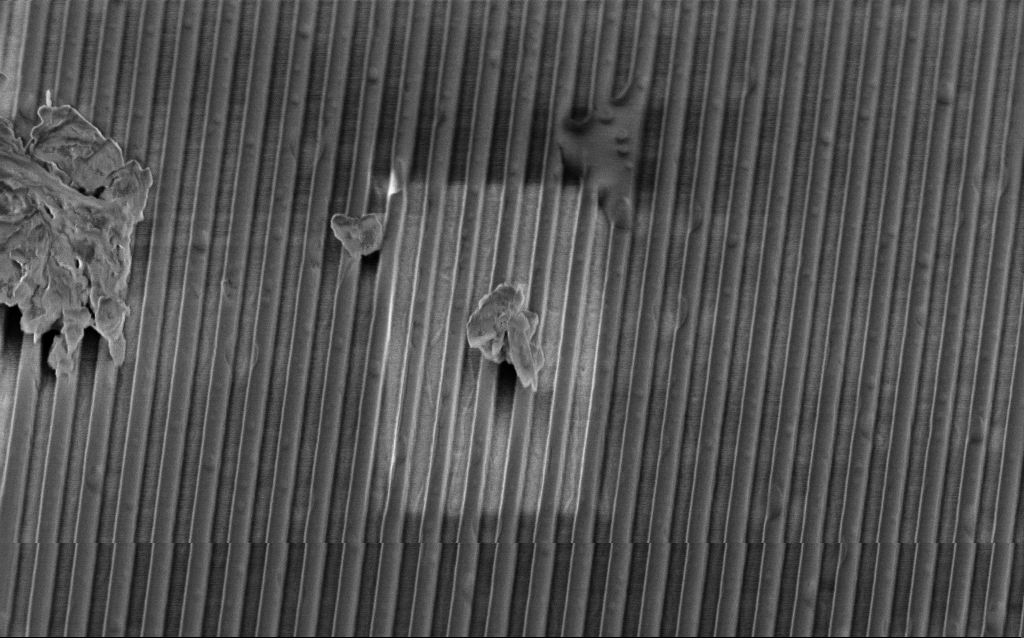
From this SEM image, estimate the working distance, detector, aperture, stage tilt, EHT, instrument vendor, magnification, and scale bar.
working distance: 3.8 mm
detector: InLens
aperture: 30 µm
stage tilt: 45°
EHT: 2 kV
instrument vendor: Zeiss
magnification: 27.97 K X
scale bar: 2000 nm